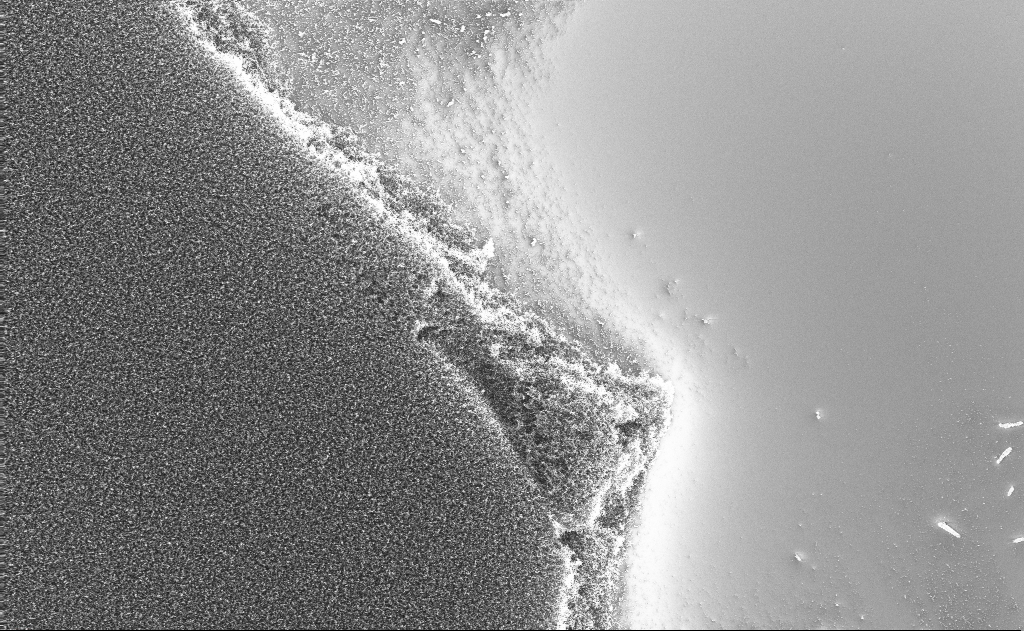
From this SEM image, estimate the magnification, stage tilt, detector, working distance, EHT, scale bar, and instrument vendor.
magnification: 1.61 K X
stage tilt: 0.6°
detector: InLens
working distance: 2 mm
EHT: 10 kV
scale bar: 20000 nm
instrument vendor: Zeiss